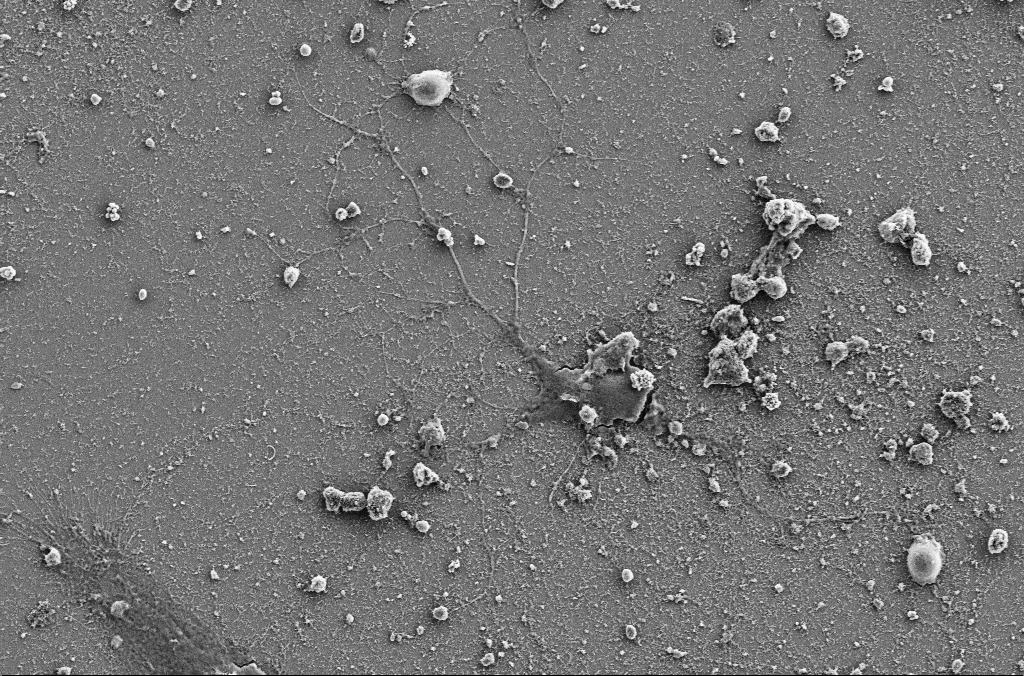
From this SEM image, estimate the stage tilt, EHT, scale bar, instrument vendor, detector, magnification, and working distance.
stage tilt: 0°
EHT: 5 kV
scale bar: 20000 nm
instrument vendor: Zeiss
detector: SE2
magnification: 2.5 K X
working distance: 4 mm